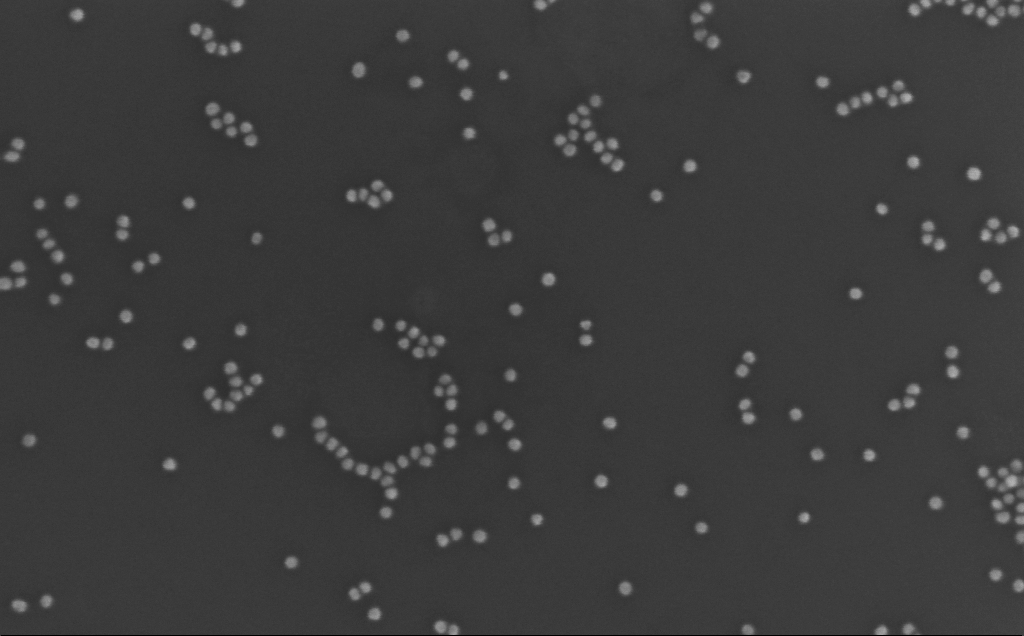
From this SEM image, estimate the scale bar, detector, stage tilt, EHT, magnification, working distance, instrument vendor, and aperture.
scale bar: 200 nm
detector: InLens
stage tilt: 0°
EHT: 10 kV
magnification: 269.95 K X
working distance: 3 mm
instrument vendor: Zeiss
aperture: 30 µm